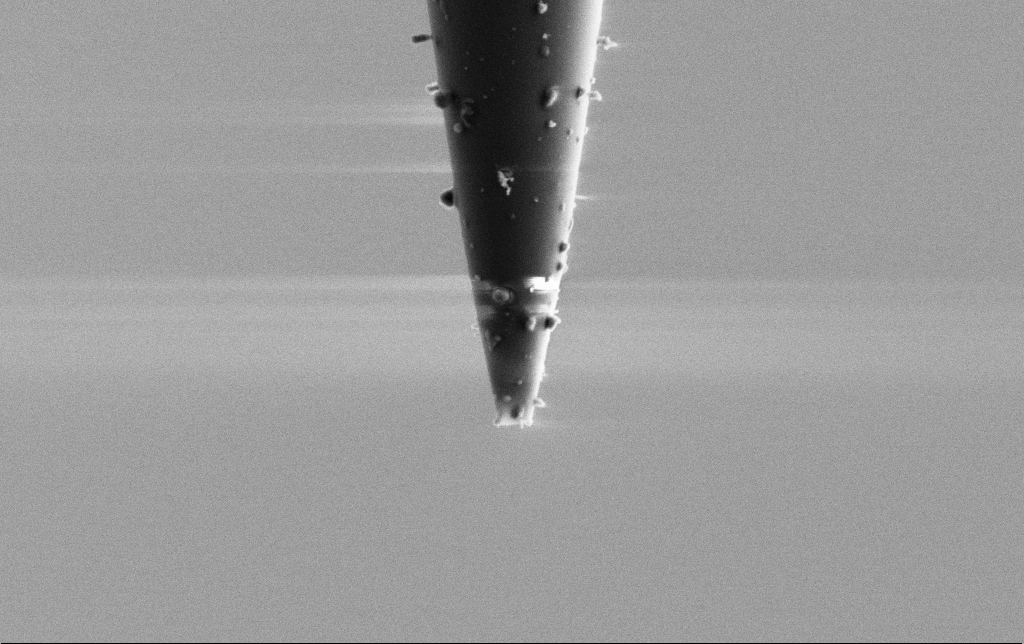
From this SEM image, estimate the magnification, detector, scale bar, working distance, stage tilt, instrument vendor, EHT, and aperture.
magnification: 25 K X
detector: SE2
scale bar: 2000 nm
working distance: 6.5 mm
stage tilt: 0°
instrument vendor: Zeiss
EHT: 2 kV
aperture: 30 µm